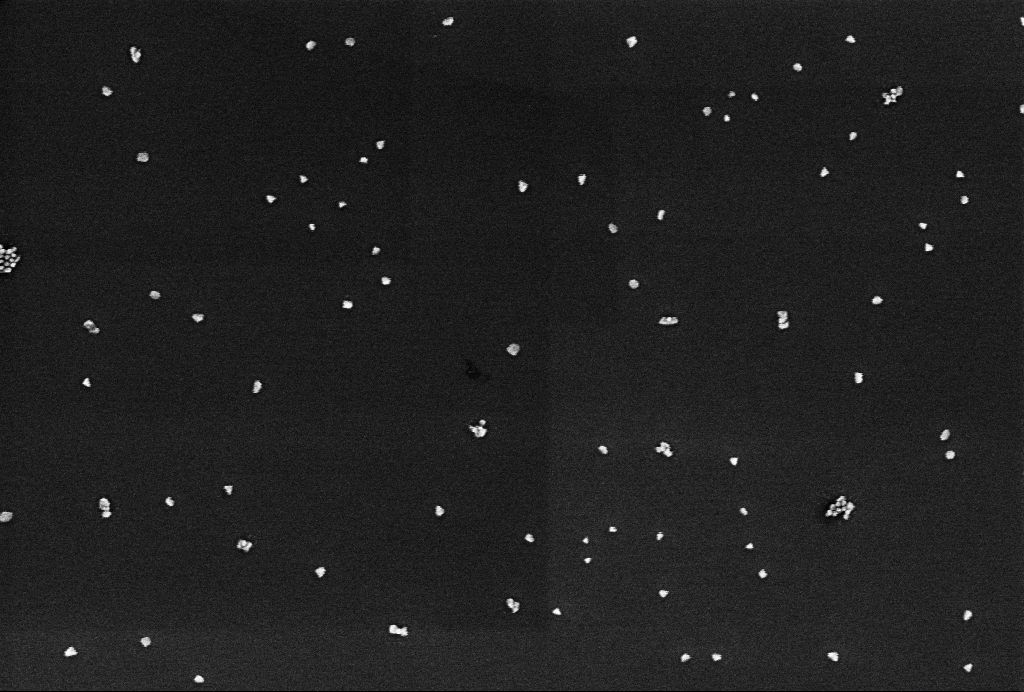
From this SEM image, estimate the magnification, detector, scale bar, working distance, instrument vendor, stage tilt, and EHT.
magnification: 86.59 K X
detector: InLens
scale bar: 200 nm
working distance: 3.4 mm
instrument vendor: Zeiss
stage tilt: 0°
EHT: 1 kV